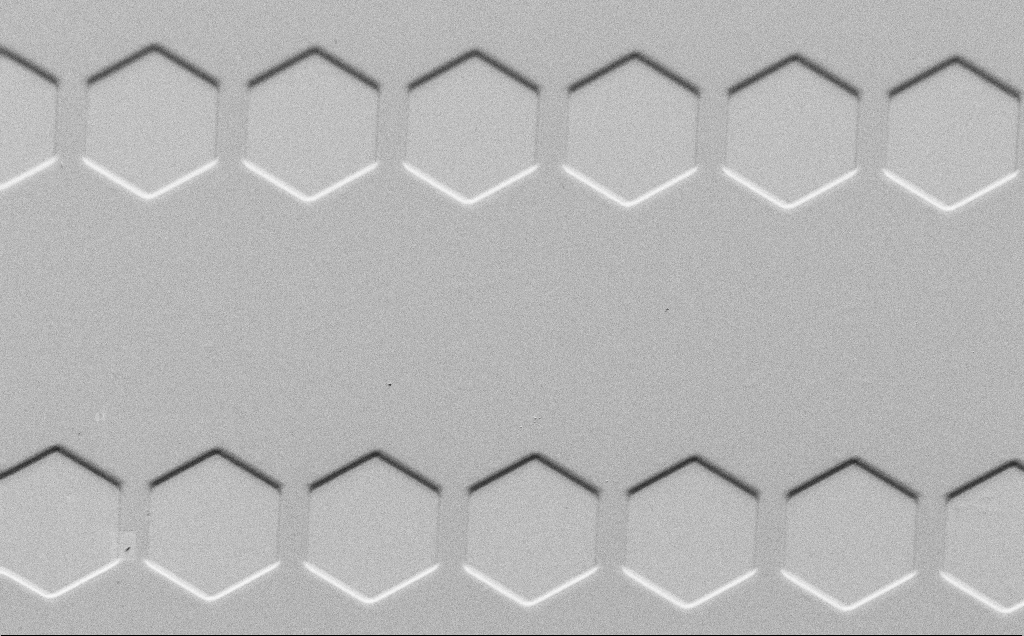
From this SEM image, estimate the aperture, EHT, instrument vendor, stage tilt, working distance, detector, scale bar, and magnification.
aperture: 30 µm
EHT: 1.5 kV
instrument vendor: Zeiss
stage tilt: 45°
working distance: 4 mm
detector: SE2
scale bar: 20000 nm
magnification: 0.994 K X